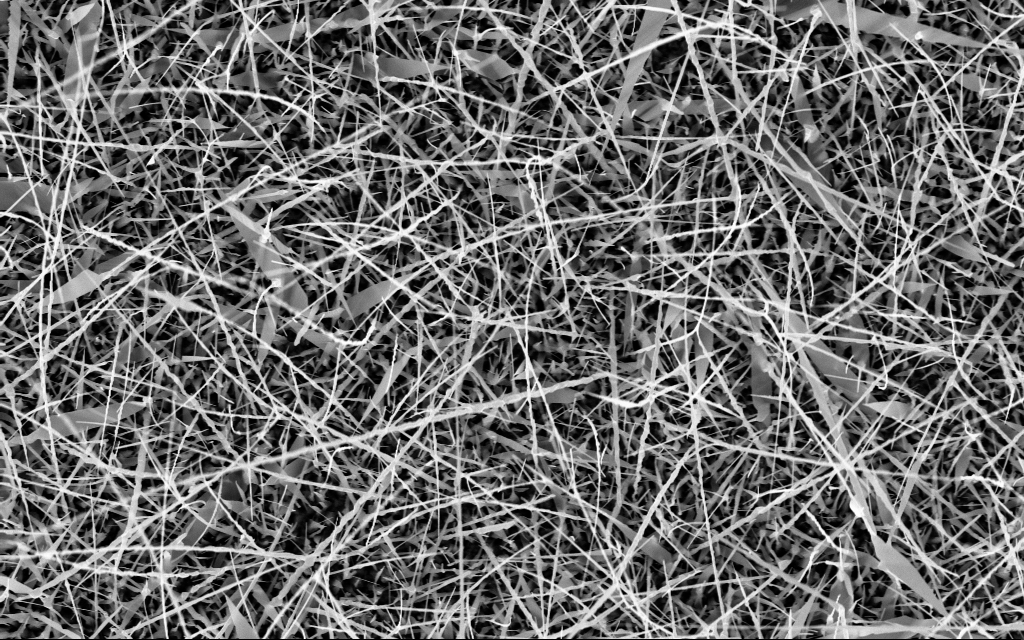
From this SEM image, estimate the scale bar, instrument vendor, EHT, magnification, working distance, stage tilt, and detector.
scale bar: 2000 nm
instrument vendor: Zeiss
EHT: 10 kV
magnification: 10 K X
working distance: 6 mm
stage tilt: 0°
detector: InLens